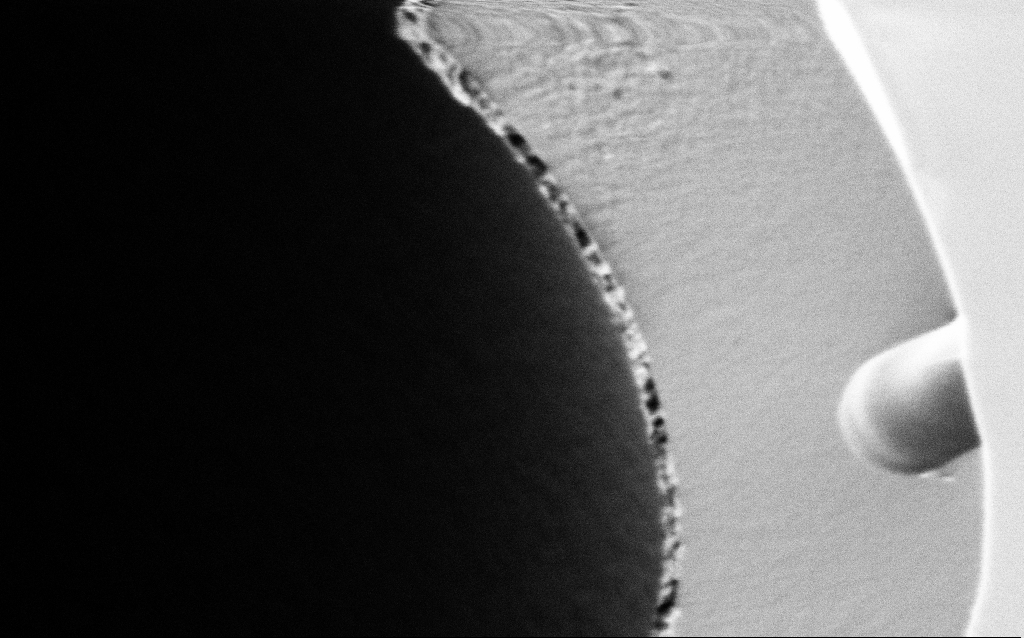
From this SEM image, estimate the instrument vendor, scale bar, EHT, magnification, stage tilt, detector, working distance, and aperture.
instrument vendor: Zeiss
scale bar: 200 nm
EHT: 1 kV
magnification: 75 K X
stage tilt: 45°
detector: SE2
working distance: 7.7 mm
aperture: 30 µm